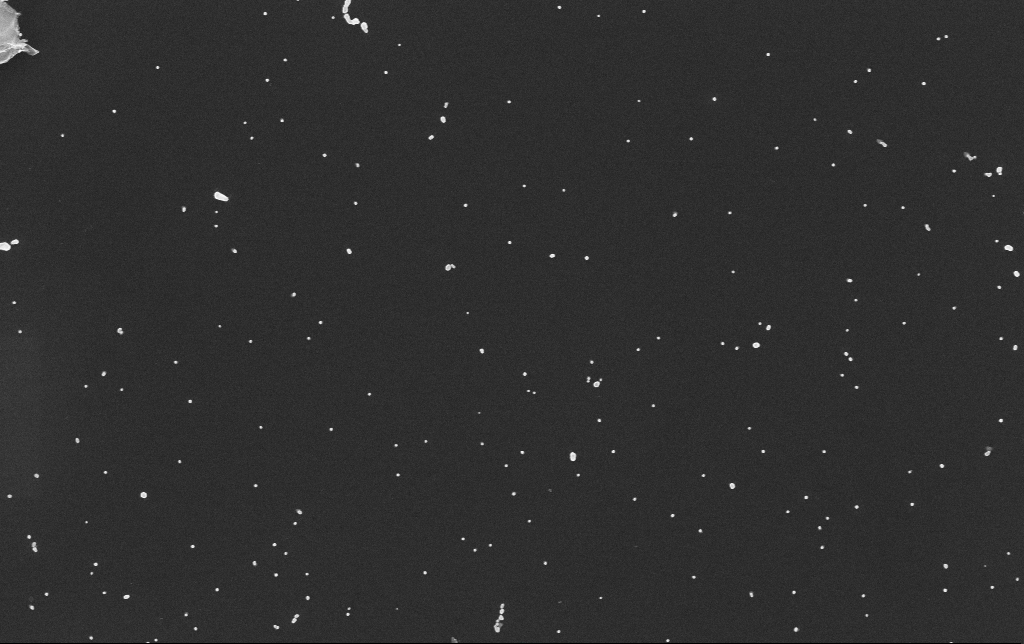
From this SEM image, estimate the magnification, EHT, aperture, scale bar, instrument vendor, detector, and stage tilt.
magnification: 50 K X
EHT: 10 kV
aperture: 30 µm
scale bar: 1000 nm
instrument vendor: Zeiss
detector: InLens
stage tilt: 0°